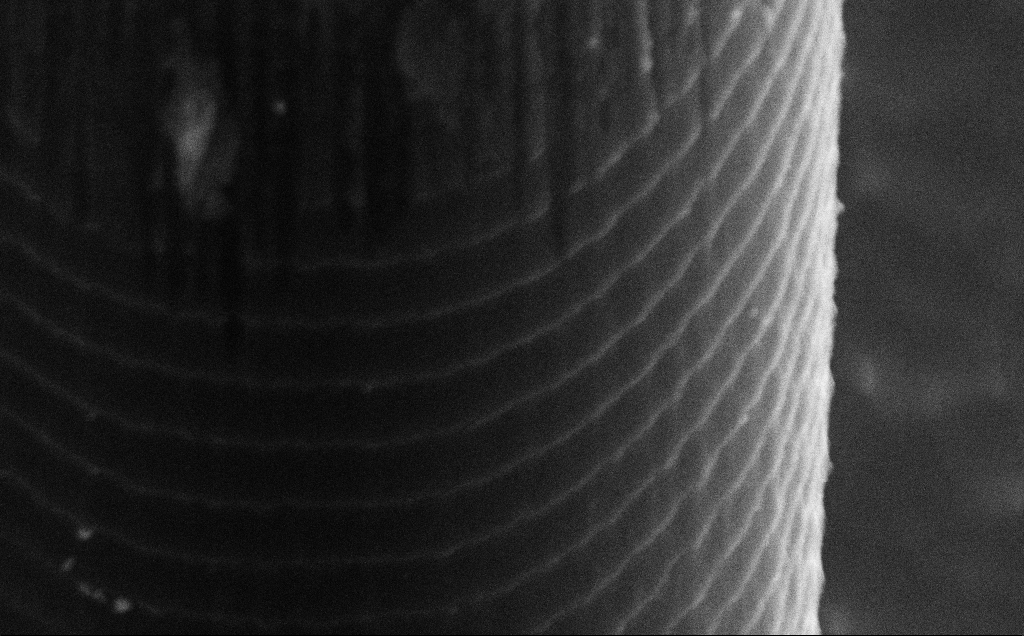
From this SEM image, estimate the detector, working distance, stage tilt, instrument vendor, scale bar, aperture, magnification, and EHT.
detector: SE2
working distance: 10 mm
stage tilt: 50°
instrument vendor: Zeiss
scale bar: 1000 nm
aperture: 30 µm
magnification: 59.14 K X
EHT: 15 kV